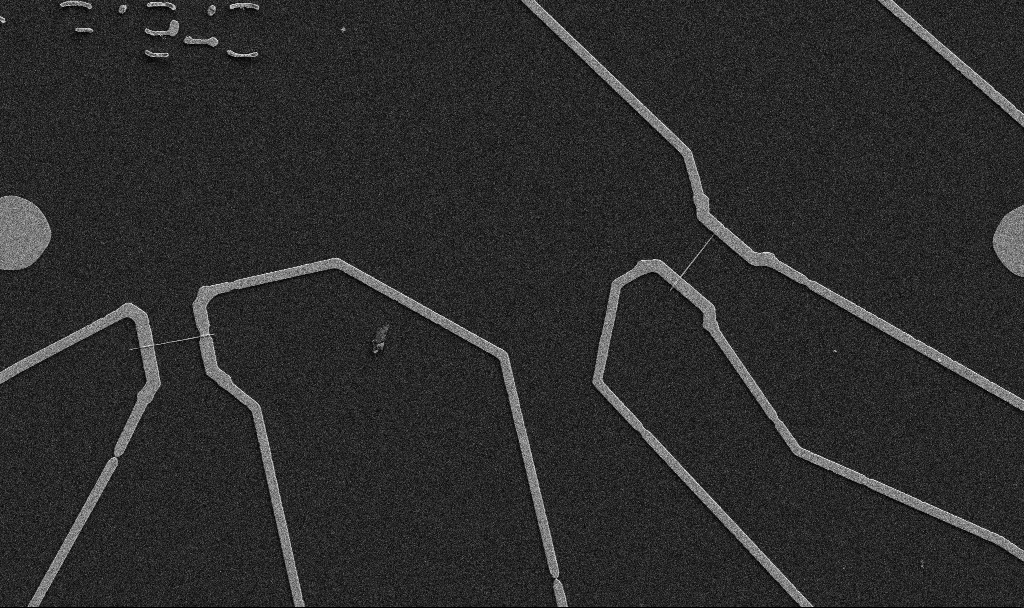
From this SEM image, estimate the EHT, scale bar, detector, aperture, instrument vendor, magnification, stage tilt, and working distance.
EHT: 5 kV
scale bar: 10000 nm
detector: SE2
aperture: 30 µm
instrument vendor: Zeiss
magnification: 5 K X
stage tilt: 0°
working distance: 10.7 mm